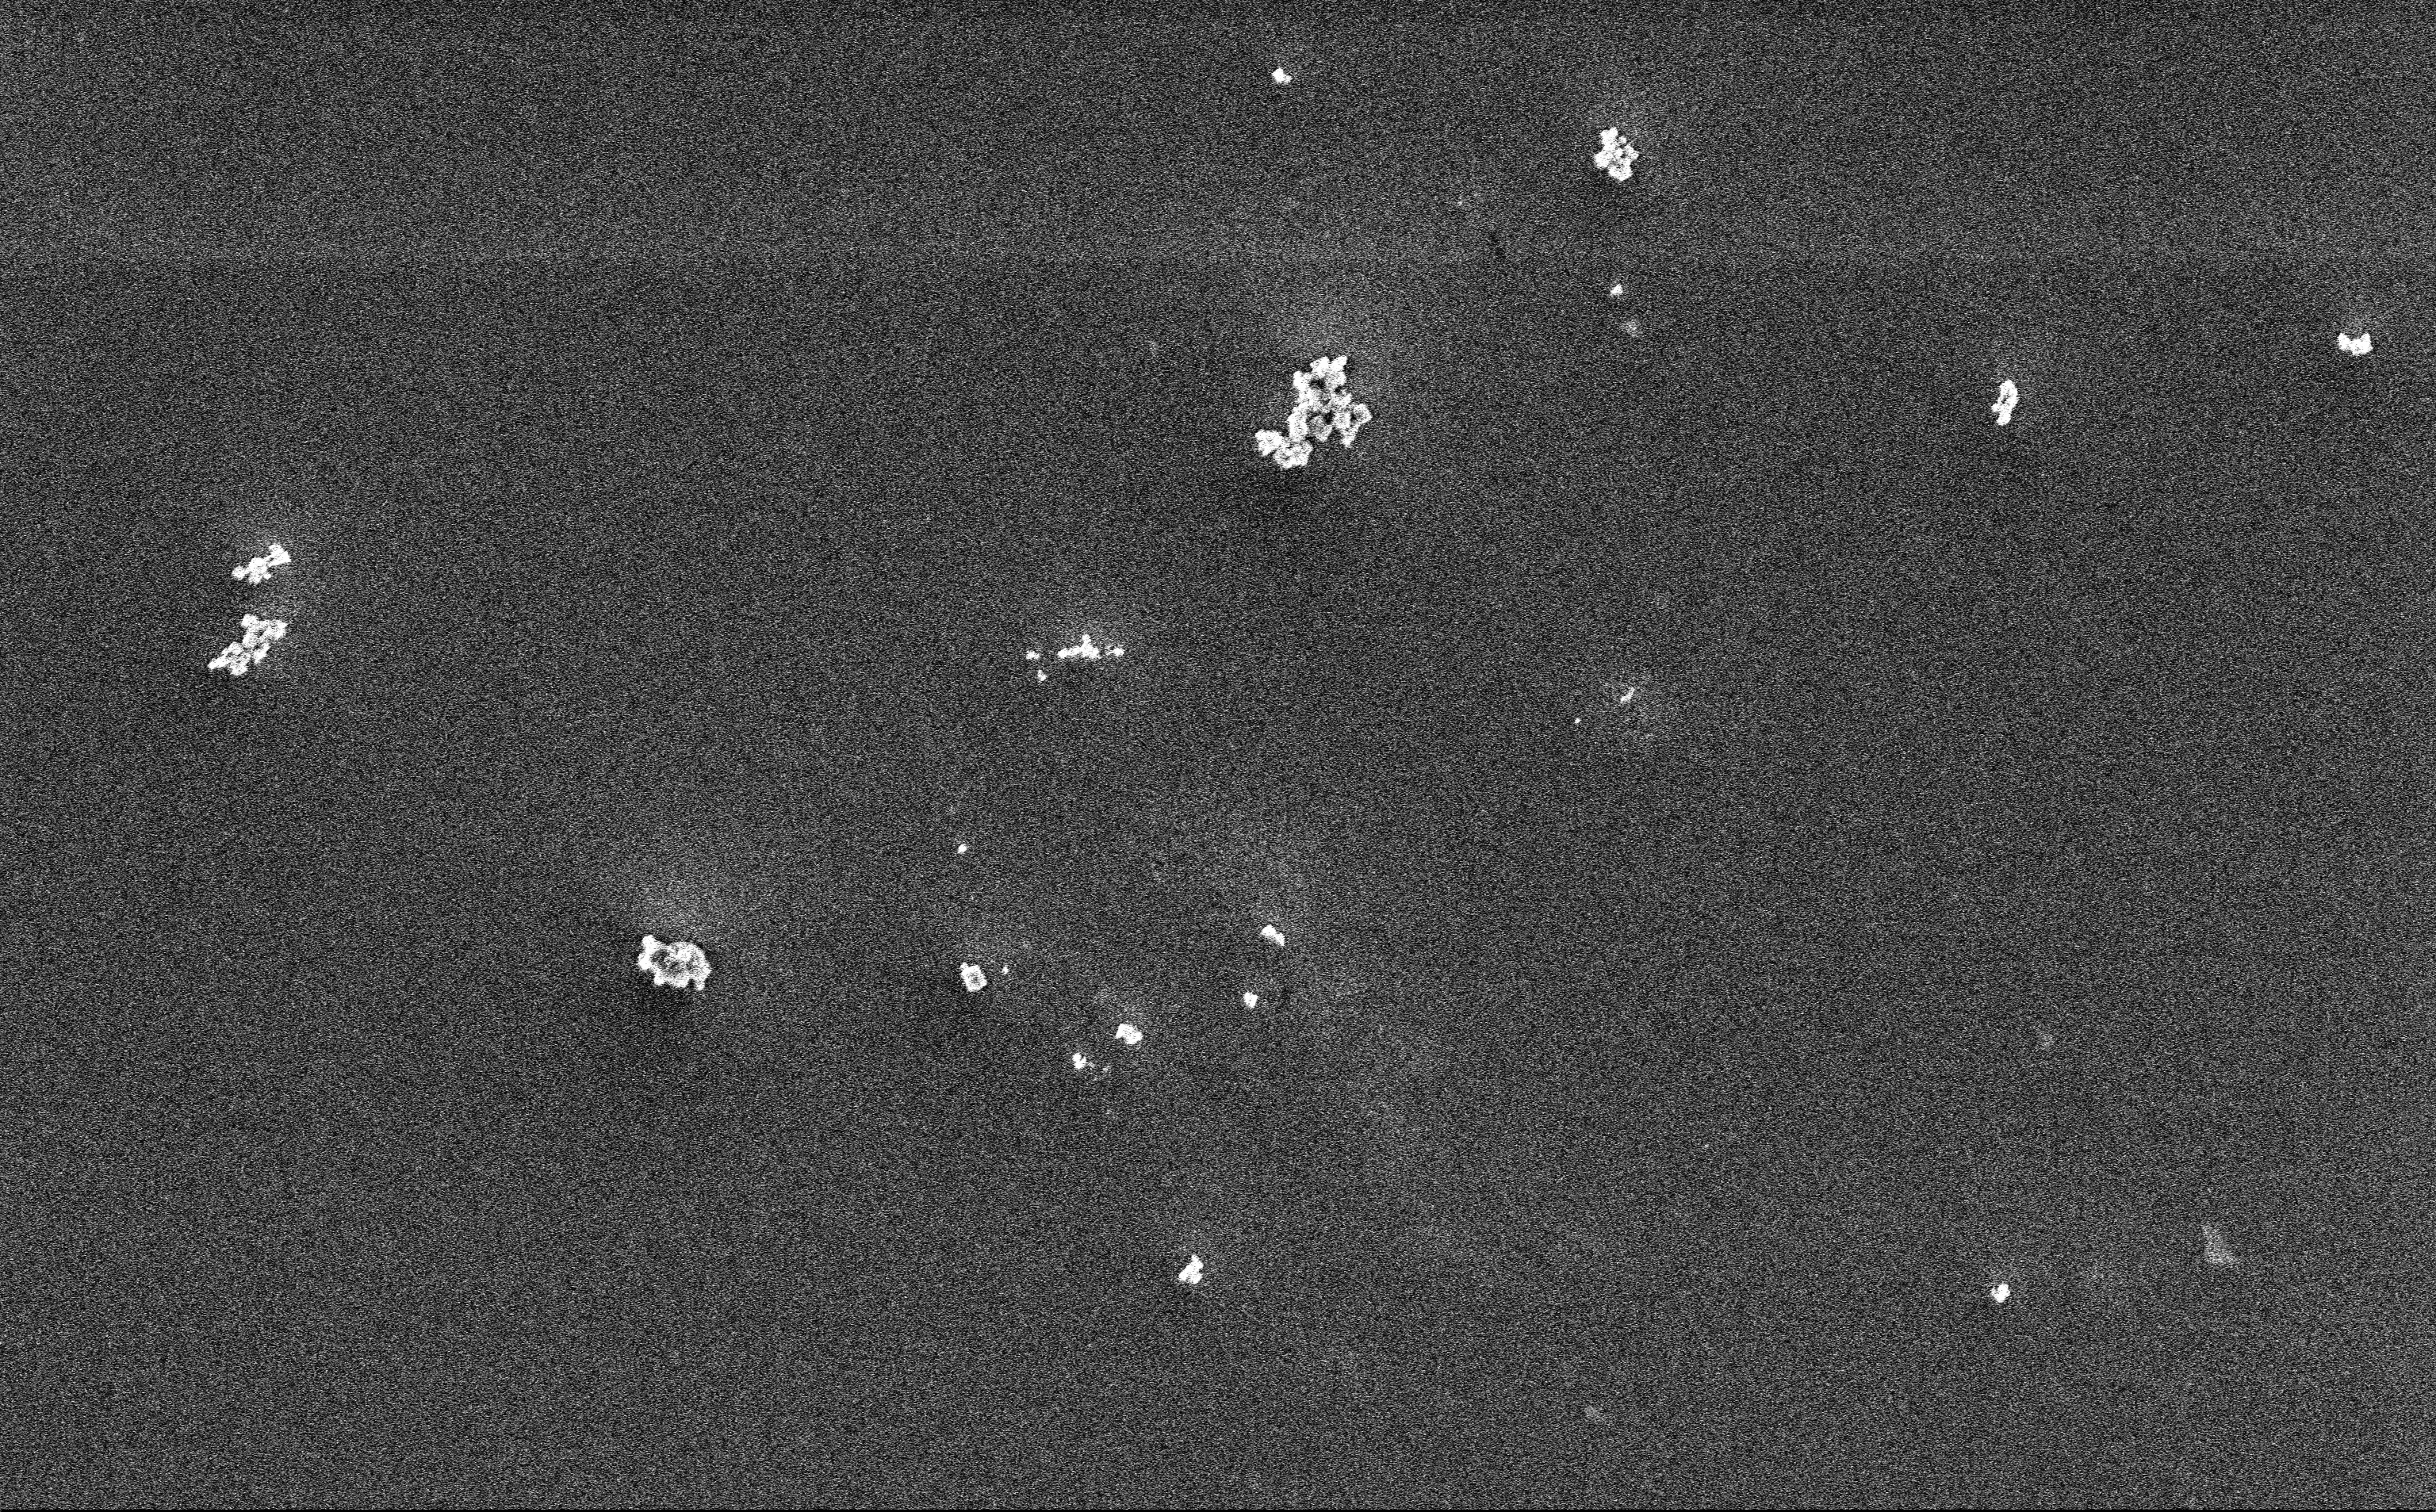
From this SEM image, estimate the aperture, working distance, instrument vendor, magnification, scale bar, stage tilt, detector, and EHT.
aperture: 30 µm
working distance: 3 mm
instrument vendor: Zeiss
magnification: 12.85 K X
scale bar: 2000 nm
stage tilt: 0°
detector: InLens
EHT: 3 kV